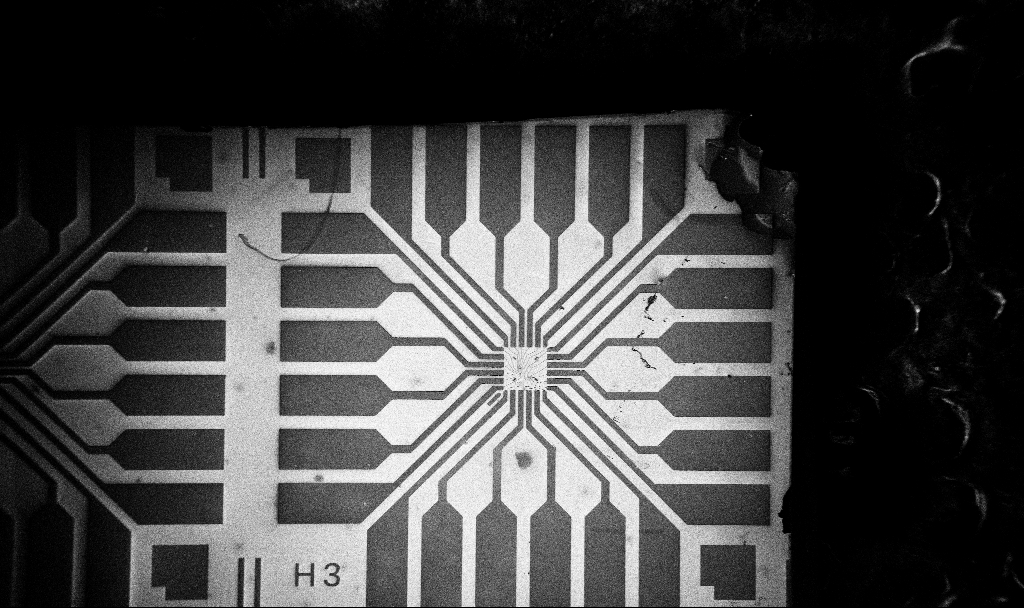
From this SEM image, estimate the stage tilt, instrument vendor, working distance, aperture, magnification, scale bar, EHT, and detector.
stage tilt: -0°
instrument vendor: Zeiss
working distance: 10.7 mm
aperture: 30 µm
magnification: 0.1 K X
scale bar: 200000 nm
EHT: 5 kV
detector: InLens